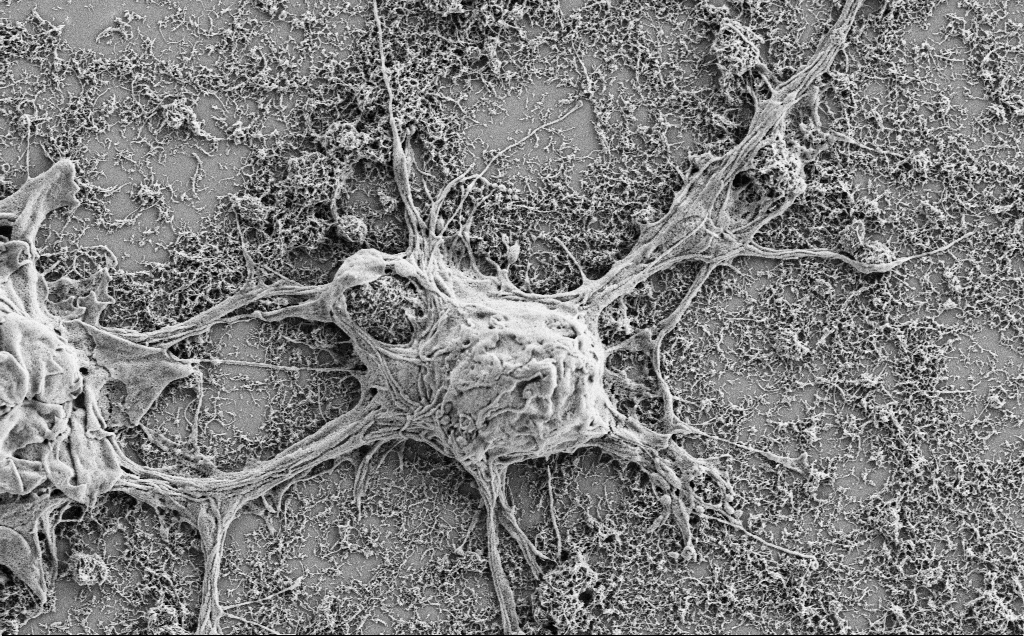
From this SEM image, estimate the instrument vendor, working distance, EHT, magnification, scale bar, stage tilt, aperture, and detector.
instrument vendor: Zeiss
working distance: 7 mm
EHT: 2 kV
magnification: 10 K X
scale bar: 2000 nm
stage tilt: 0°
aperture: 30 µm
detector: SE2